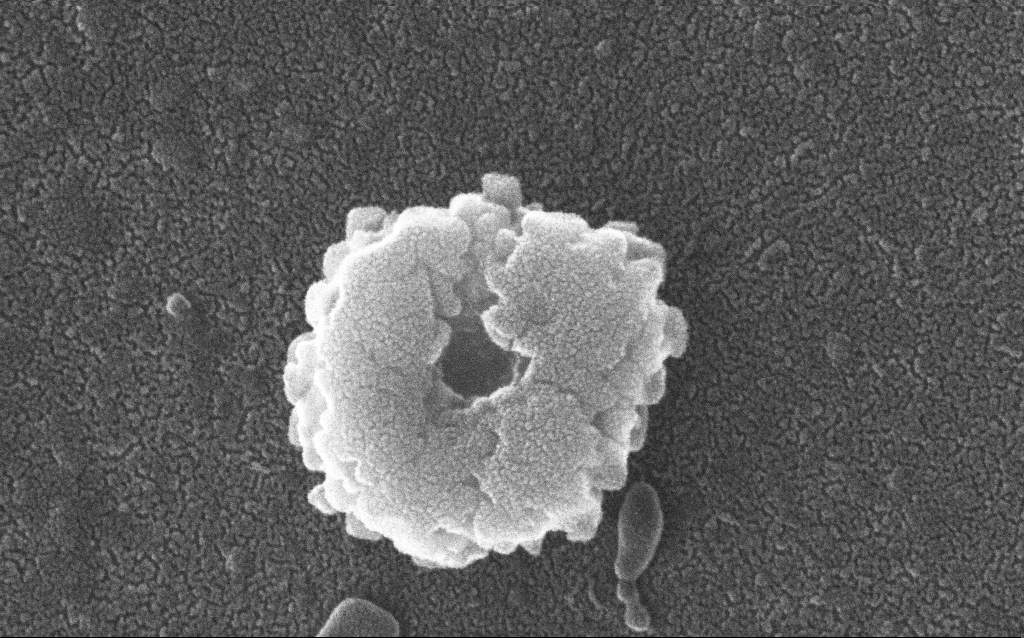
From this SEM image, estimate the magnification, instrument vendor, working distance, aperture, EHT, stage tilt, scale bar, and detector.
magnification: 300 K X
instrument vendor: Zeiss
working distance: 1.5 mm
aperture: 30 µm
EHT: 20 kV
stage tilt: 0°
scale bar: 200 nm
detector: InLens